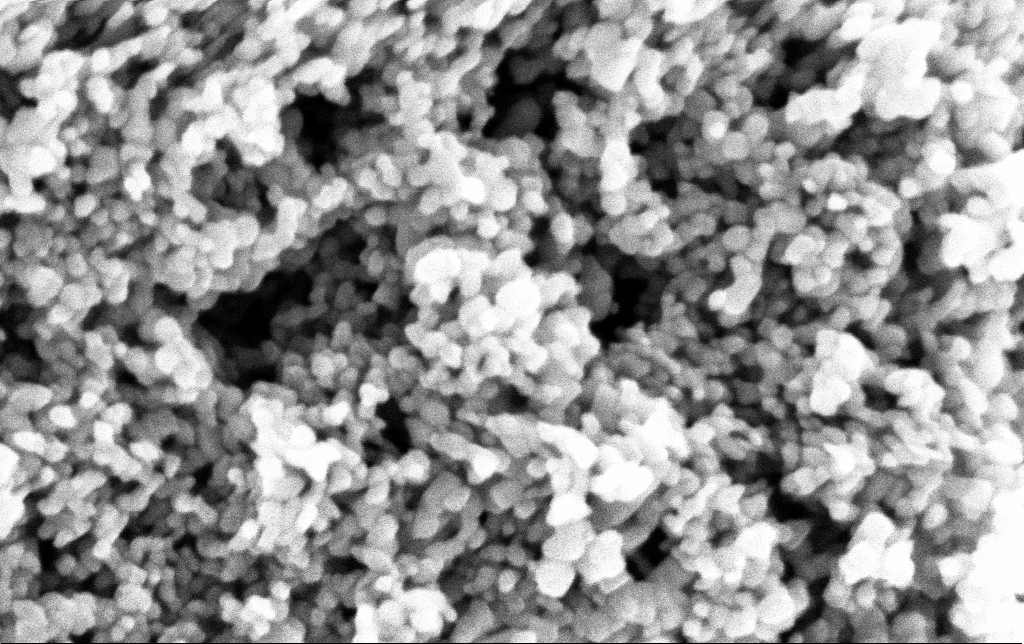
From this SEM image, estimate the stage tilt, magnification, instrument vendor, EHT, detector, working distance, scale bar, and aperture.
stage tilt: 0°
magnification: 282.84 K X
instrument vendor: Zeiss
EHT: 3 kV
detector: InLens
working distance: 2.5 mm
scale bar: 200 nm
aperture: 30 µm